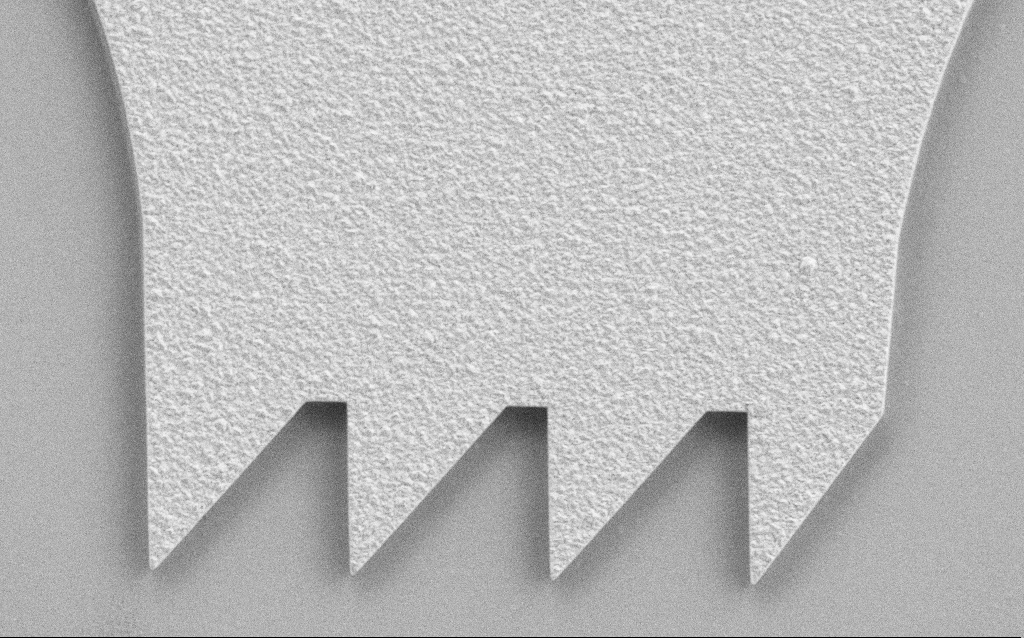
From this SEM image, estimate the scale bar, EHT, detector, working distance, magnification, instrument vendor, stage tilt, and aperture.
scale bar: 10000 nm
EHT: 3 kV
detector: SE2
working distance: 4.5 mm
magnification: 6.5 K X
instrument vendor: Zeiss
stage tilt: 0°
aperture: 30 µm